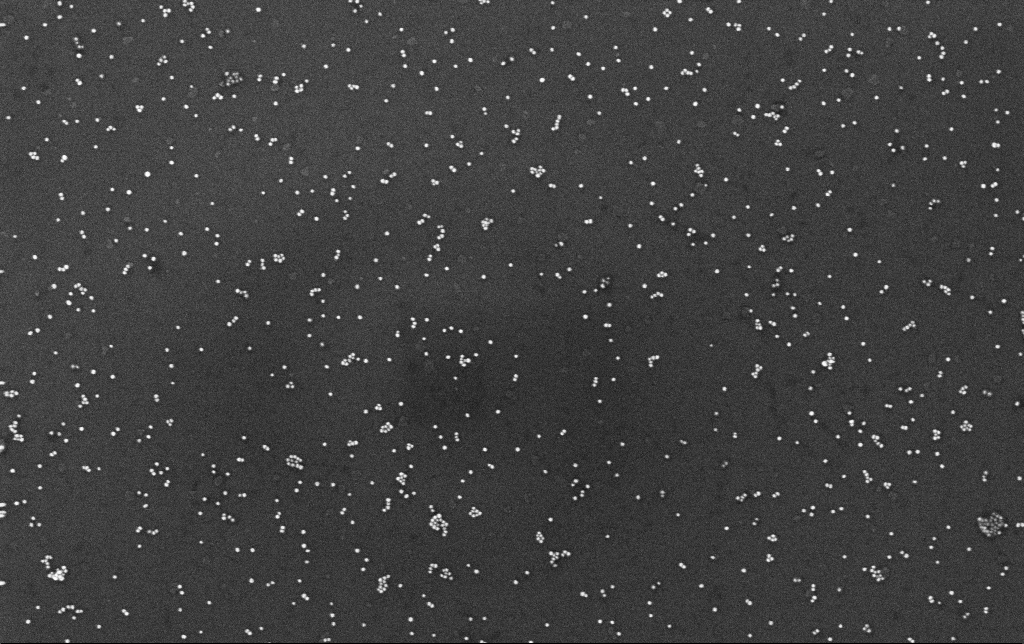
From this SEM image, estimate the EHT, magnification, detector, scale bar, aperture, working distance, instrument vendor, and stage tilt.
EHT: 10 kV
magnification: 100 K X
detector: InLens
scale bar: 200 nm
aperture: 30 µm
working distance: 3.4 mm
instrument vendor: Zeiss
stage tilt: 0°